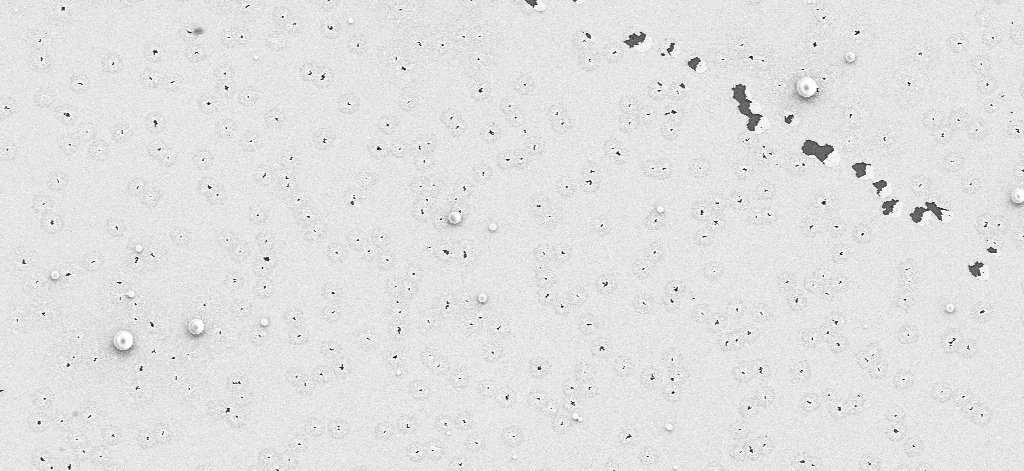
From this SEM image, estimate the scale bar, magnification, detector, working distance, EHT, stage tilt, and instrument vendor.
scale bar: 10000 nm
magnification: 4.2 K X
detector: SE2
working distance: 12 mm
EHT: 5 kV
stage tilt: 0°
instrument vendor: Zeiss